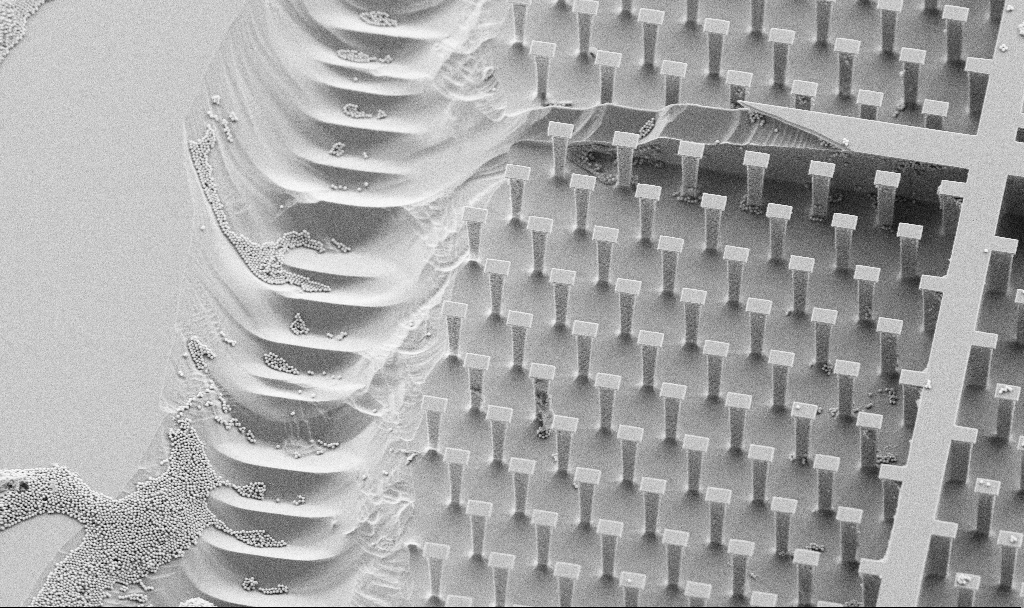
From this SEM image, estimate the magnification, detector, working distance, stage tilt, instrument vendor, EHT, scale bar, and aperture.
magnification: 2.03 K X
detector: SE2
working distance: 4.6 mm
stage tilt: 30°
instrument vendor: Zeiss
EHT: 5 kV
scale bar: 10000 nm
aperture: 30 µm